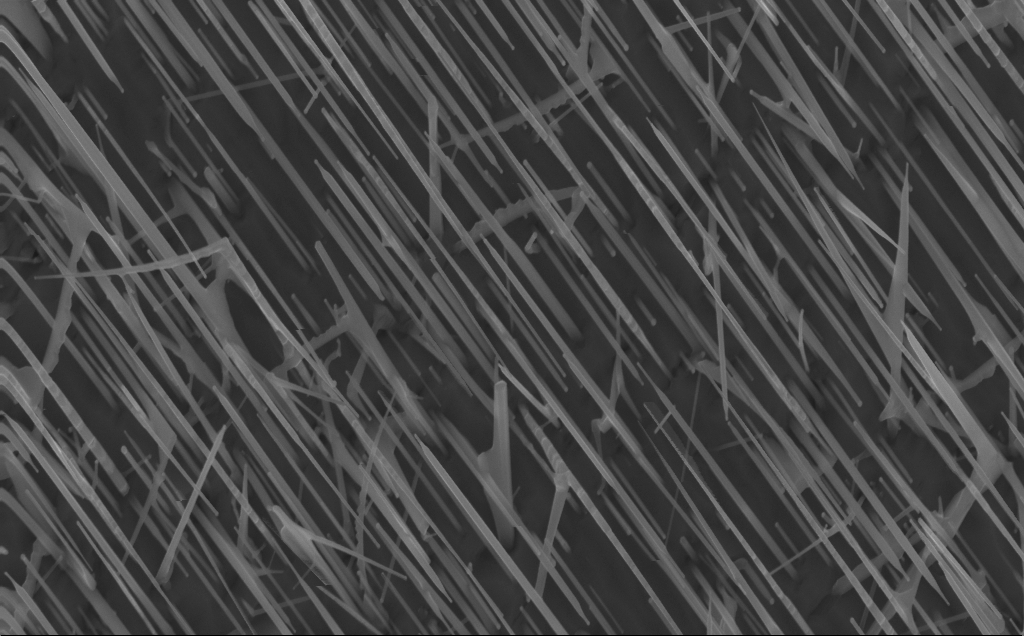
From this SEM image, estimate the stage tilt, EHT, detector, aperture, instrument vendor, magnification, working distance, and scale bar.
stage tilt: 0°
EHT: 10 kV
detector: InLens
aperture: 30 µm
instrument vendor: Zeiss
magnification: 40 K X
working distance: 4 mm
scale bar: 1000 nm